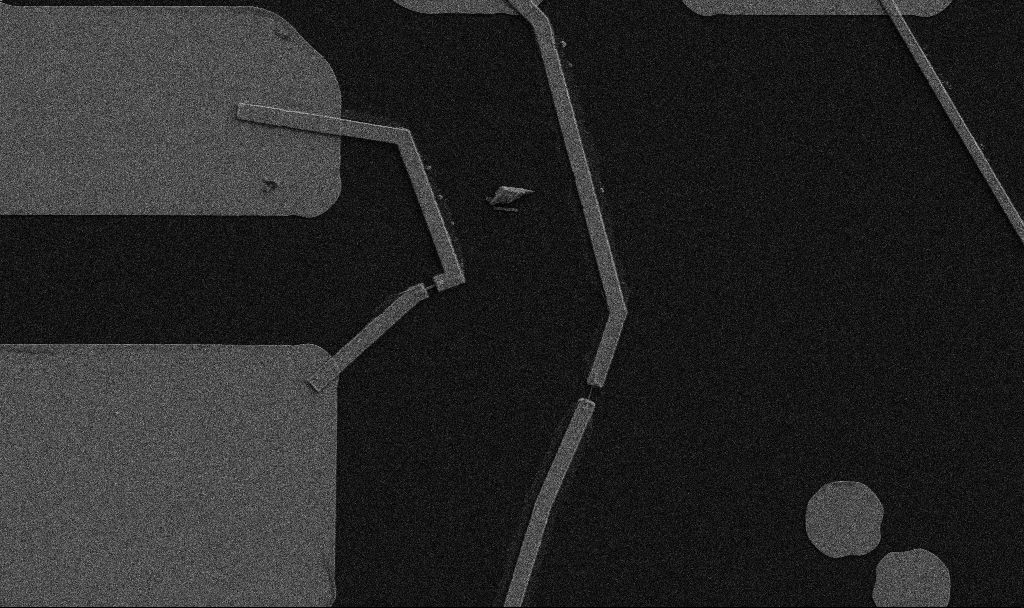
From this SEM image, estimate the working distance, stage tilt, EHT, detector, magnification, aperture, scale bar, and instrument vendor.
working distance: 10.7 mm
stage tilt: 0°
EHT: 5 kV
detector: SE2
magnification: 5 K X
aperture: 30 µm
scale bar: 10000 nm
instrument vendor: Zeiss